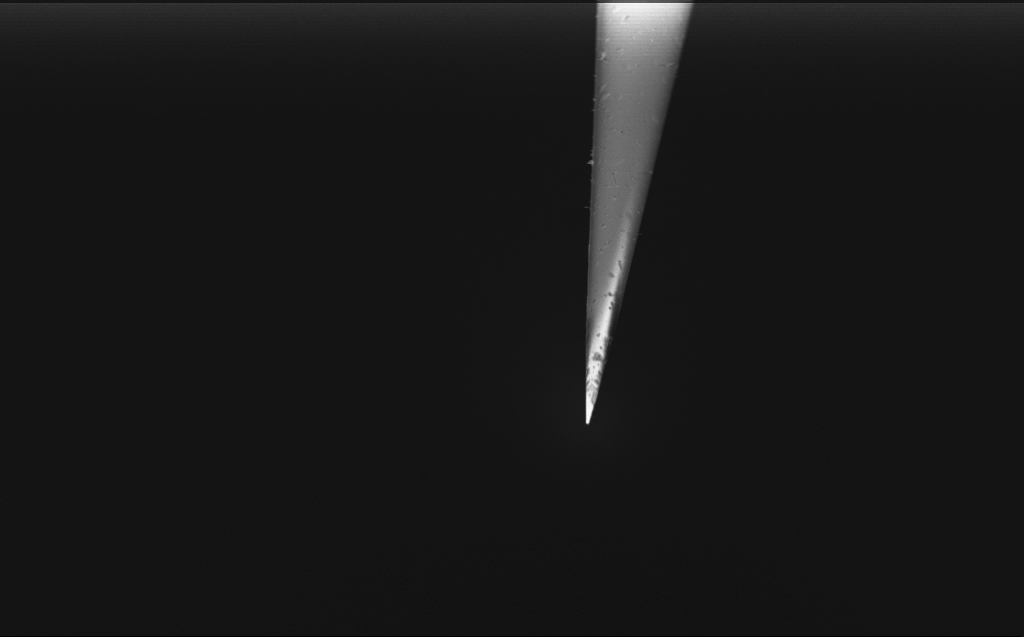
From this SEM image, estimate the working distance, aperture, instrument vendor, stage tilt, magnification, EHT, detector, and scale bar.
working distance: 4 mm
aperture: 30 µm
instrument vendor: Zeiss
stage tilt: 45°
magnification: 4.84 K X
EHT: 2 kV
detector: InLens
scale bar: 10000 nm